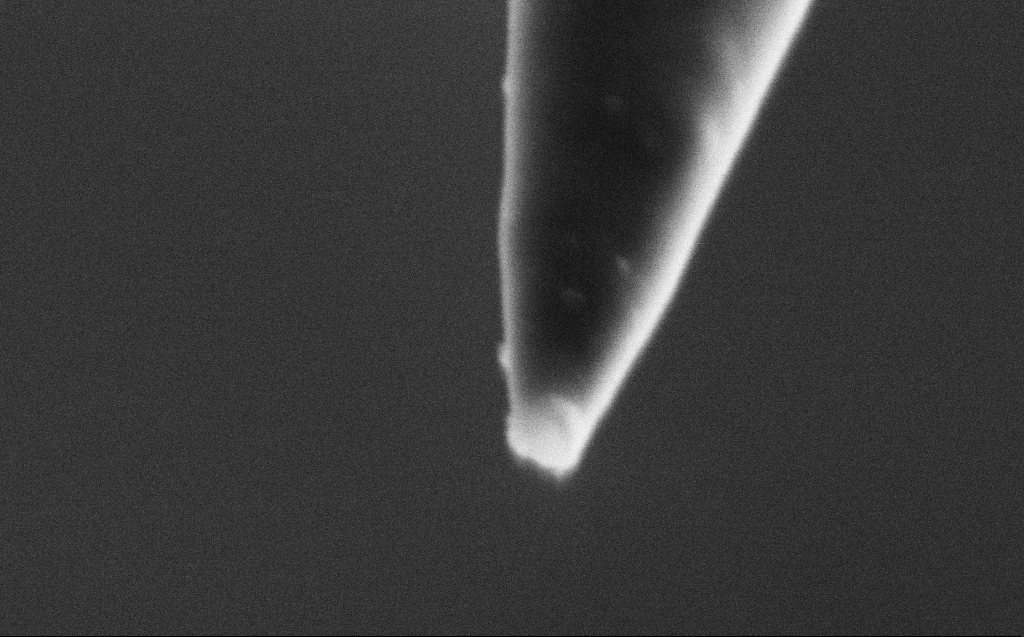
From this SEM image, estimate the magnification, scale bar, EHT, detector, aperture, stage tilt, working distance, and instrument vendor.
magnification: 250 K X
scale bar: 200 nm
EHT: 2 kV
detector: SE2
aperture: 30 µm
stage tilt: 45°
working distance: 4 mm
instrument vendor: Zeiss